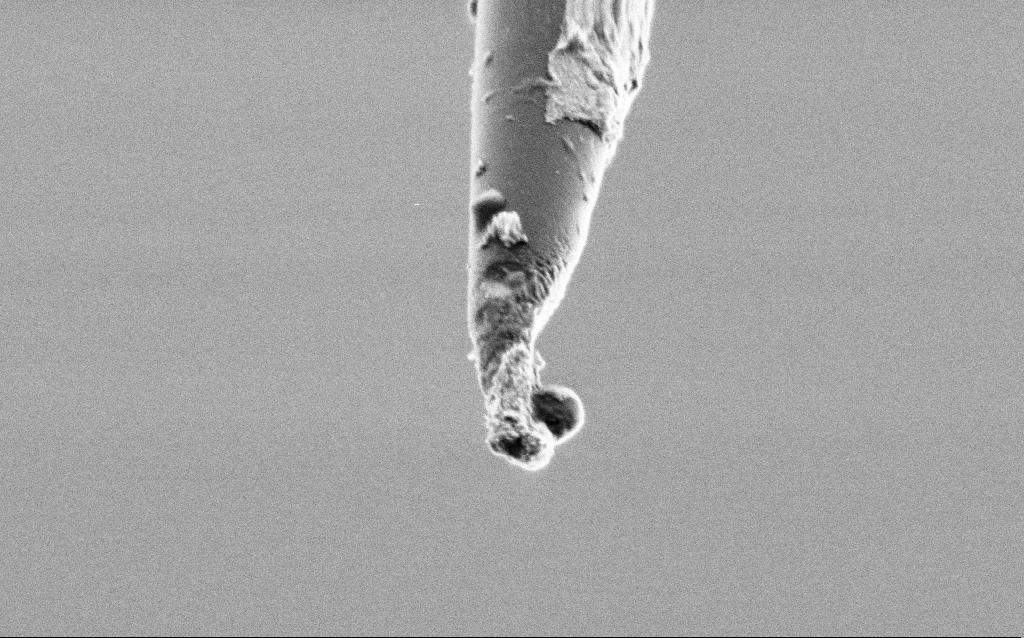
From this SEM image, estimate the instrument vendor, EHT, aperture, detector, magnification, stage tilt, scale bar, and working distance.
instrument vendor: Zeiss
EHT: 1 kV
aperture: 30 µm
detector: SE2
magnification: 50 K X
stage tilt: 45°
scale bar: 1000 nm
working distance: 6.8 mm